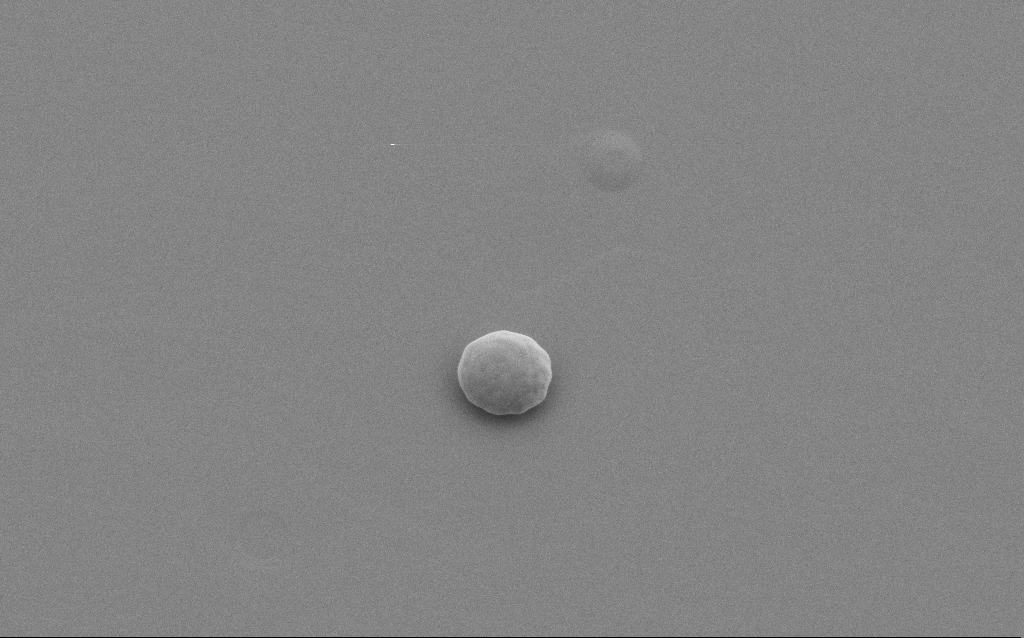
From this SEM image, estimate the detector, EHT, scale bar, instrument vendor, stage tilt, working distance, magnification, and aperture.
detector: SE2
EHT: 5 kV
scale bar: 2000 nm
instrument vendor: Zeiss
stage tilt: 20°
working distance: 4 mm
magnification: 13.37 K X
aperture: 30 µm